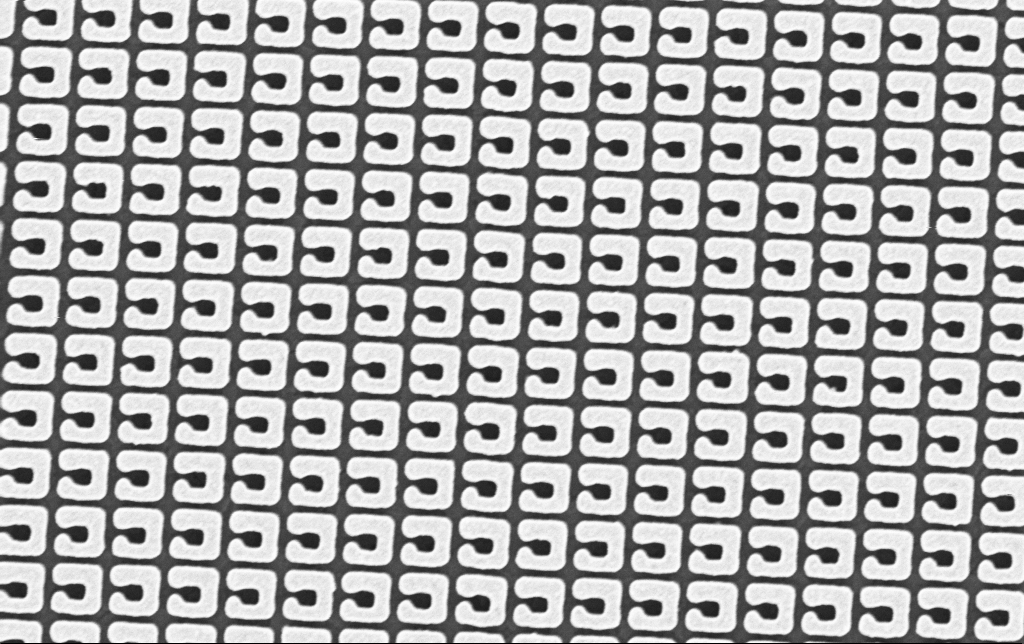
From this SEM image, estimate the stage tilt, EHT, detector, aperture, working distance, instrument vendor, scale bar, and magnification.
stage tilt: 0°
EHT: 3 kV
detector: InLens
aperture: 30 µm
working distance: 3.6 mm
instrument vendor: Zeiss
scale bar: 1000 nm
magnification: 46.18 K X